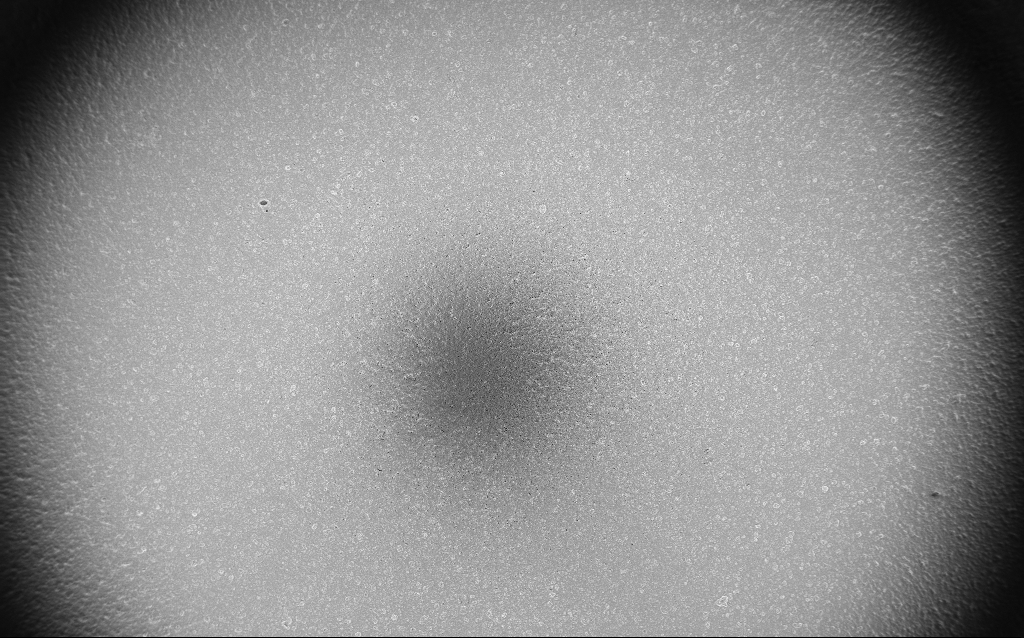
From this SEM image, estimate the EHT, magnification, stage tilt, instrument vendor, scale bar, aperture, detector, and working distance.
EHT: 5 kV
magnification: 0.1 K X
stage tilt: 0°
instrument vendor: Zeiss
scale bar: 200000 nm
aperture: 30 µm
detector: InLens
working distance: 3 mm